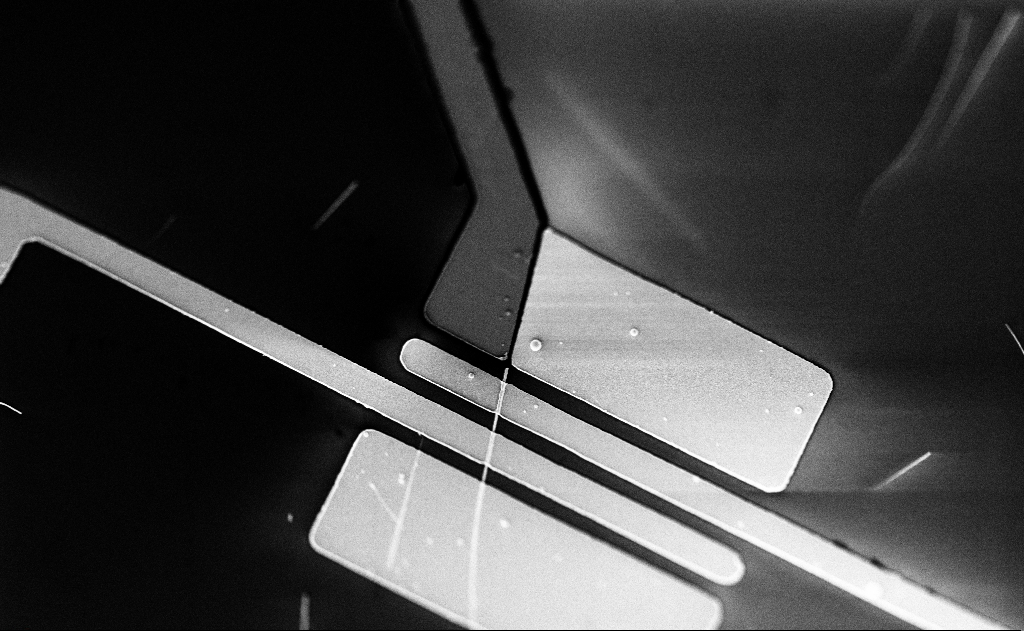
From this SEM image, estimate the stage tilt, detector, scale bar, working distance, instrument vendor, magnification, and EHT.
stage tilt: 0°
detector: SE2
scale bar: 10000 nm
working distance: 20 mm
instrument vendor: Zeiss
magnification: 4.76 K X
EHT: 5 kV